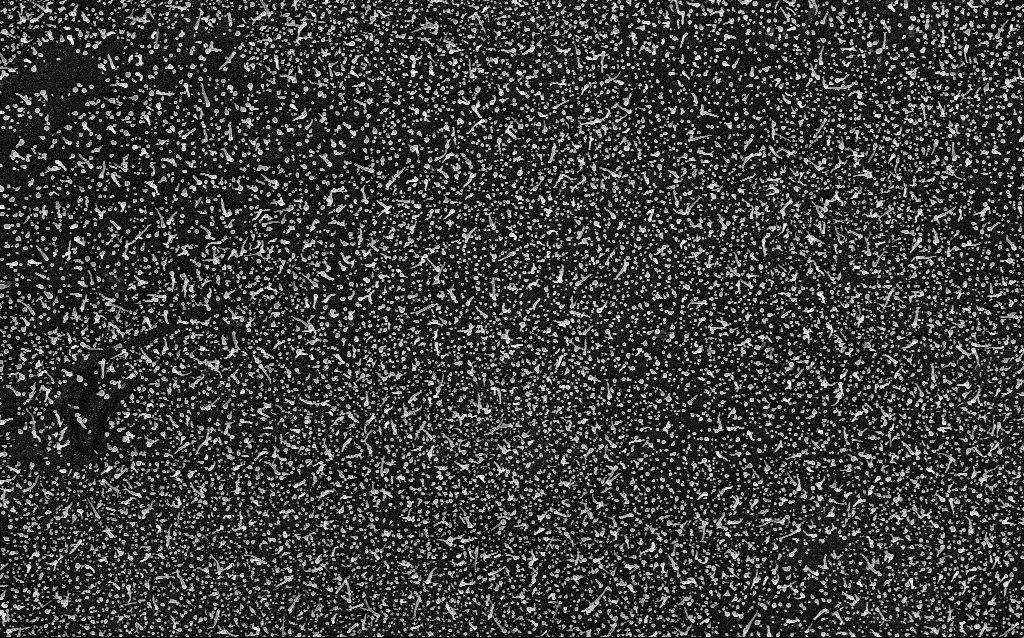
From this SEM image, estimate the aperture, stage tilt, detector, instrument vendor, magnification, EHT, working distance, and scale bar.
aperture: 30 µm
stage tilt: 0°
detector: InLens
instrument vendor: Zeiss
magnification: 10 K X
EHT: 5 kV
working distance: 1.7 mm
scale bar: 2000 nm